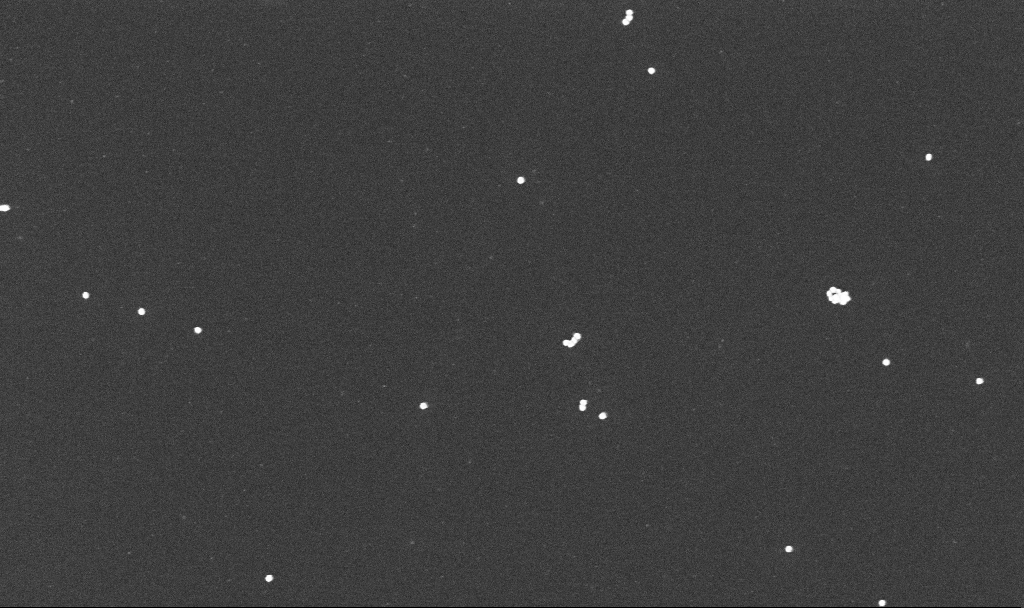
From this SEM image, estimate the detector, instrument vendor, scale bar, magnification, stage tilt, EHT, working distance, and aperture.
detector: InLens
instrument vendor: Zeiss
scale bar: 200 nm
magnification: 100 K X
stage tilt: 0°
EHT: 10 kV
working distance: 3.2 mm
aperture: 30 µm